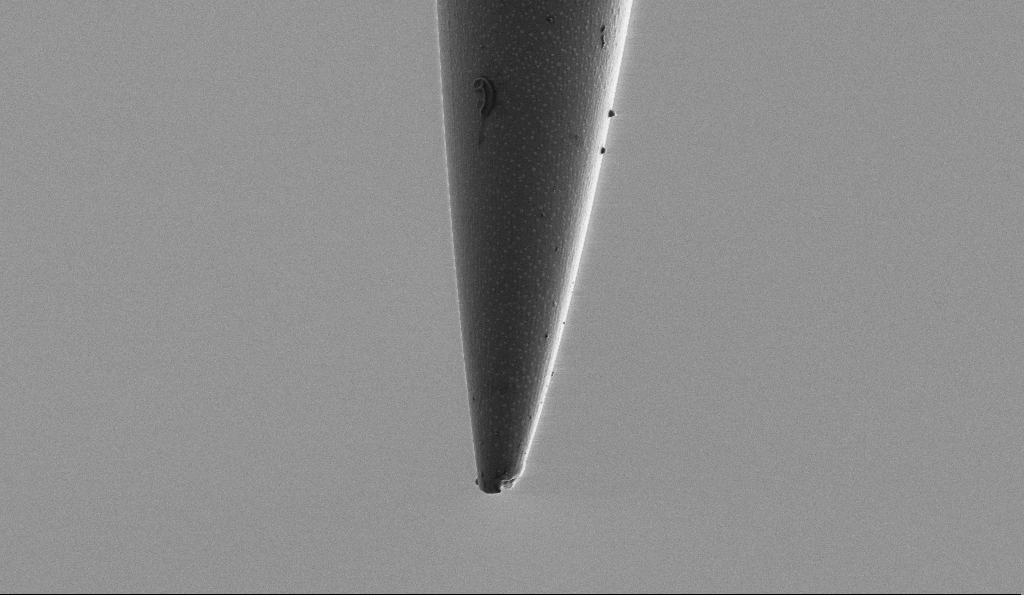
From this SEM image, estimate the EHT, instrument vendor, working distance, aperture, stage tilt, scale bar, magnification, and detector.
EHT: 1 kV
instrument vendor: Zeiss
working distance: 6.6 mm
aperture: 30 µm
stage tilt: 0°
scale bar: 2000 nm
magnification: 10 K X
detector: SE2